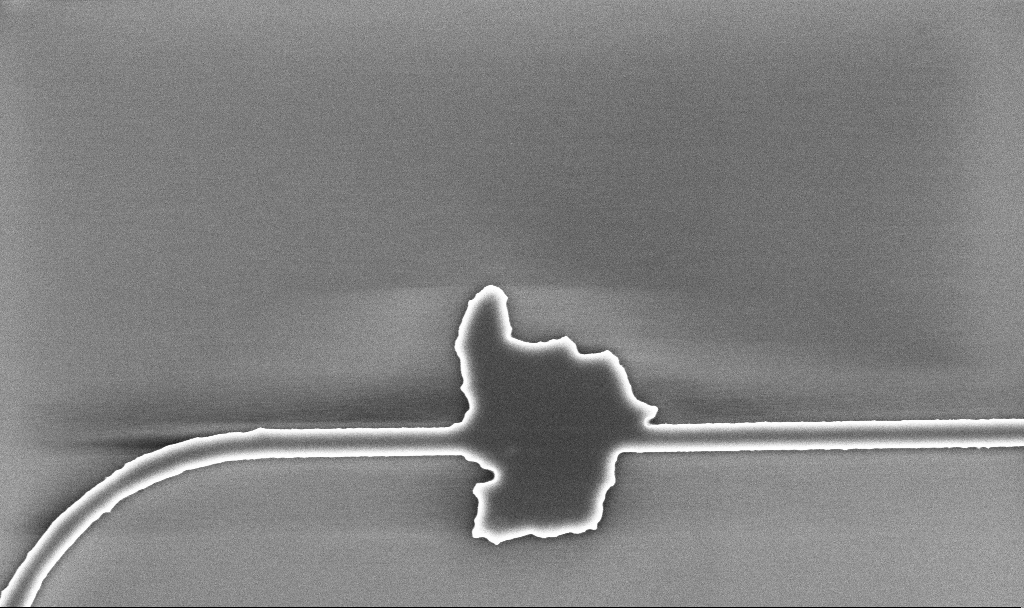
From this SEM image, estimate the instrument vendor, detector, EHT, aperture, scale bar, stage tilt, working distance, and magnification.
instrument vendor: Zeiss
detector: InLens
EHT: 5 kV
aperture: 30 µm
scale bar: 2000 nm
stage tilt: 0°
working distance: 5.2 mm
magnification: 20.88 K X